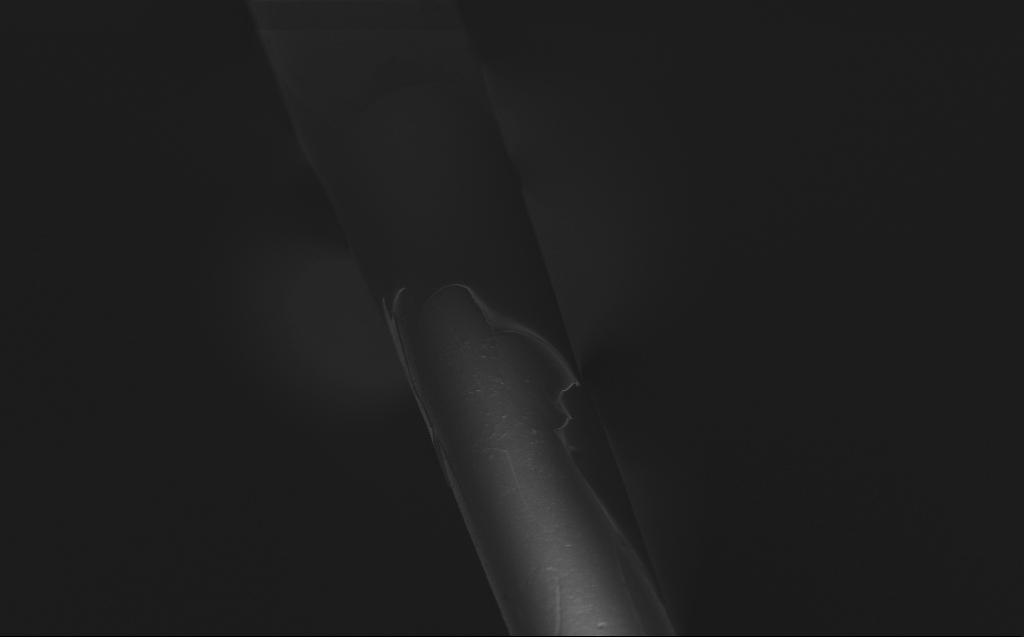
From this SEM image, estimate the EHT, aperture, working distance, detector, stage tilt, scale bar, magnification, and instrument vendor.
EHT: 1 kV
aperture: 30 µm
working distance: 4 mm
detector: InLens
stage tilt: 45°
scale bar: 10000 nm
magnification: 2.5 K X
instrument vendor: Zeiss